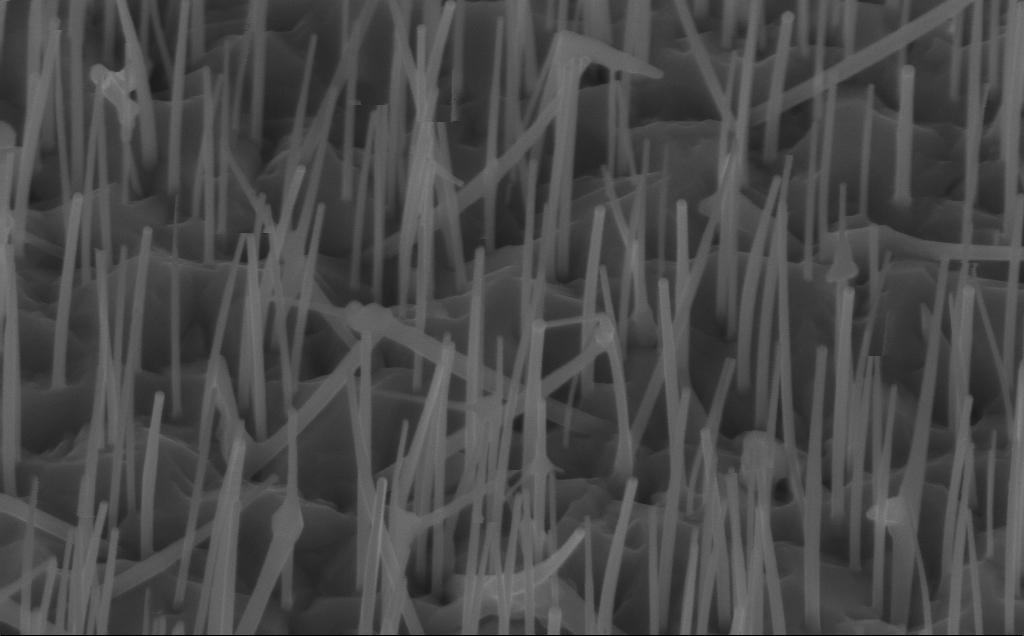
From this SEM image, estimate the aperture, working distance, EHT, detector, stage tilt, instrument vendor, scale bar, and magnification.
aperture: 30 µm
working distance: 7 mm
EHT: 10 kV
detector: InLens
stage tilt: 45°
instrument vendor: Zeiss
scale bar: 200 nm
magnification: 80 K X